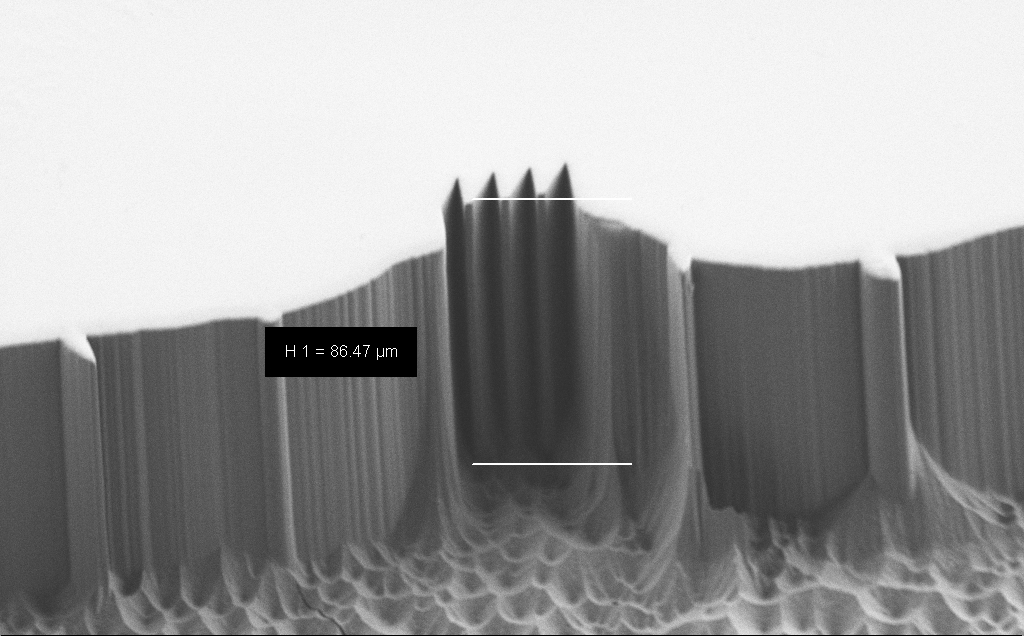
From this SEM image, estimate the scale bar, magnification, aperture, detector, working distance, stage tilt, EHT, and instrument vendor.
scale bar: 20000 nm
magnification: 1.13 K X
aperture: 30 µm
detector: SE2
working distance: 7 mm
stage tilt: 45°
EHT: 1.2 kV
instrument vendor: Zeiss